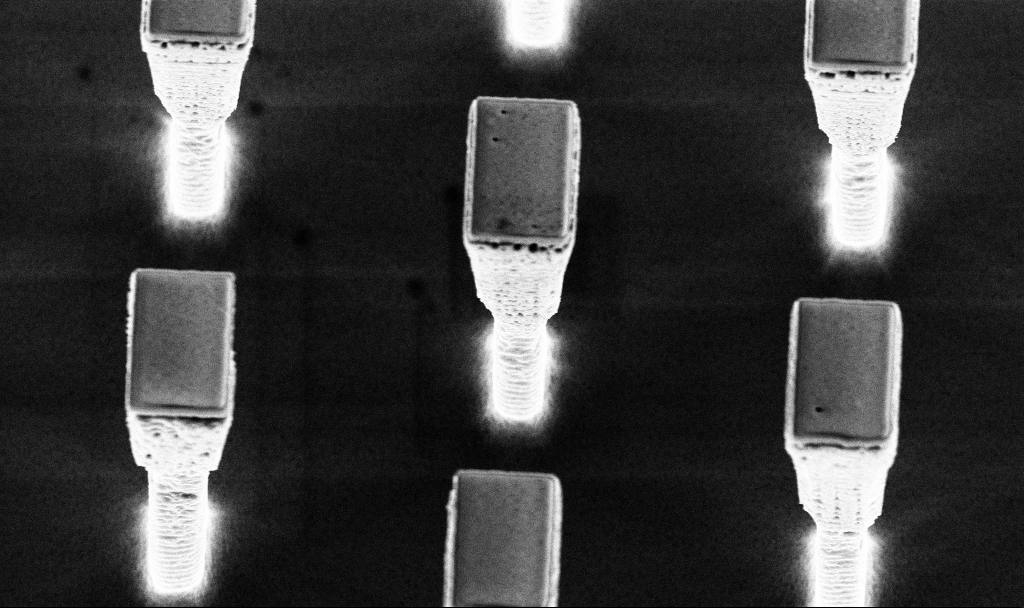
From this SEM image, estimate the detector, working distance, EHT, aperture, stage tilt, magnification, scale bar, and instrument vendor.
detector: InLens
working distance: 4.1 mm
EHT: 5 kV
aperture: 30 µm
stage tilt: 20.2°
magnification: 12.18 K X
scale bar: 2000 nm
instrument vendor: Zeiss